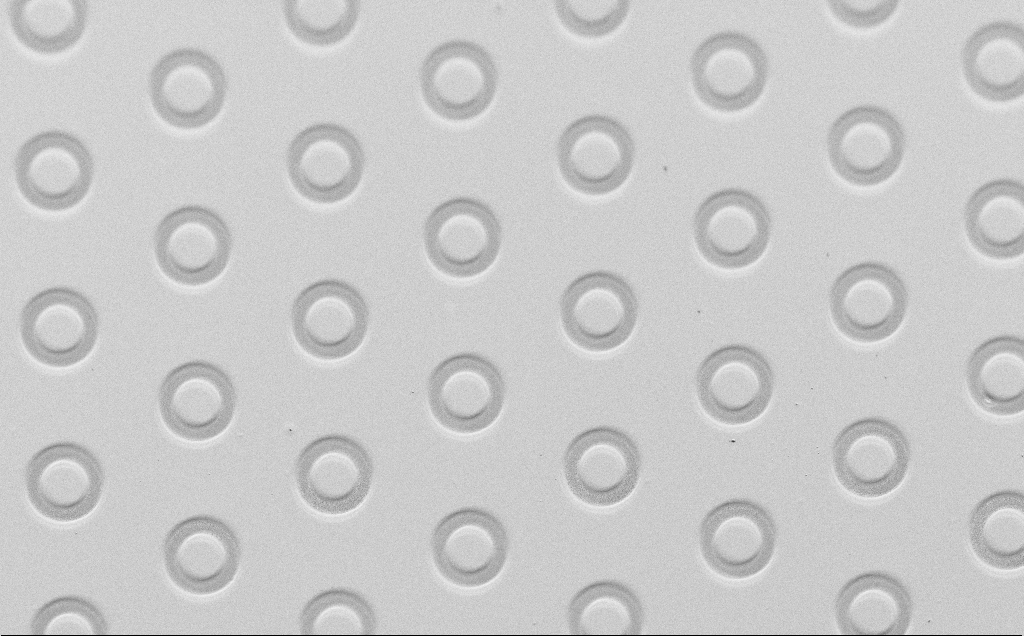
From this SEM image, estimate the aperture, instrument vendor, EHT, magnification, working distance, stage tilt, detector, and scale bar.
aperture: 30 µm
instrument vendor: Zeiss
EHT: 1.5 kV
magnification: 0.583 K X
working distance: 4 mm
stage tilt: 45°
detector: SE2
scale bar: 100000 nm